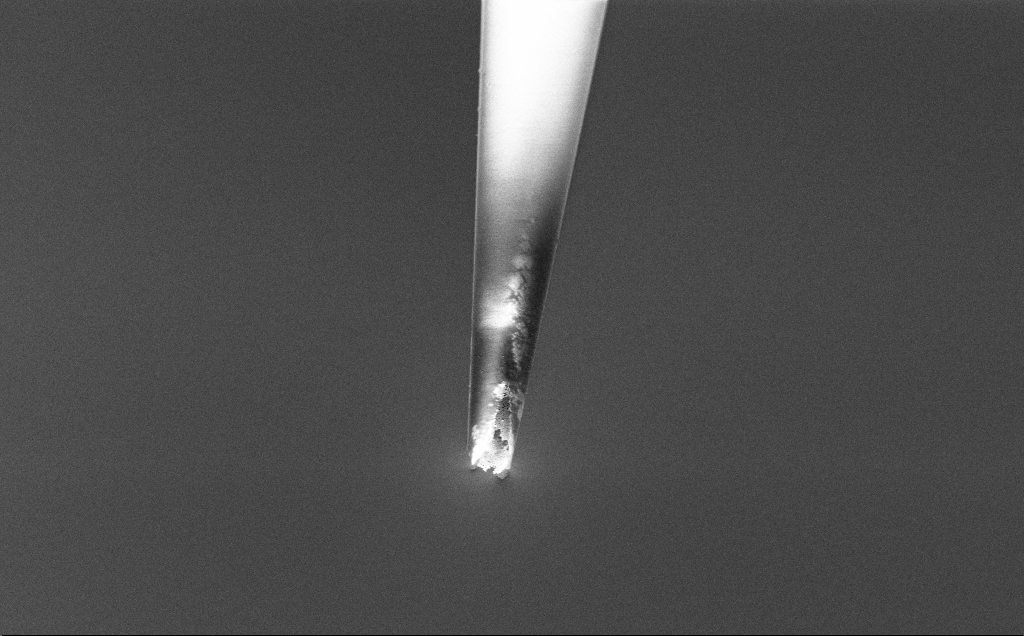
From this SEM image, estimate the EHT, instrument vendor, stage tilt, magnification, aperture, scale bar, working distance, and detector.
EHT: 3 kV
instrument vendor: Zeiss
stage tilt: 45°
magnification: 5 K X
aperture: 30 µm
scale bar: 10000 nm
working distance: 7.4 mm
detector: InLens